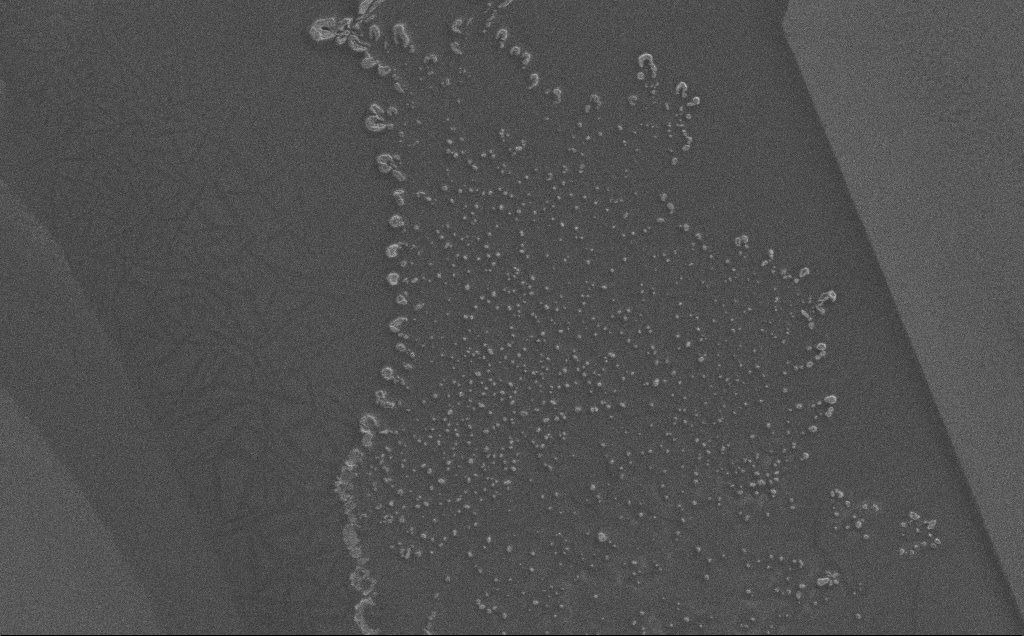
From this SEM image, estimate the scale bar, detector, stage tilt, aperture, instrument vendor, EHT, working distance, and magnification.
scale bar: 10000 nm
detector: SE2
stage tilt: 0°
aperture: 30 µm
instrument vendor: Zeiss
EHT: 0.5 kV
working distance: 3 mm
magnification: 2.73 K X